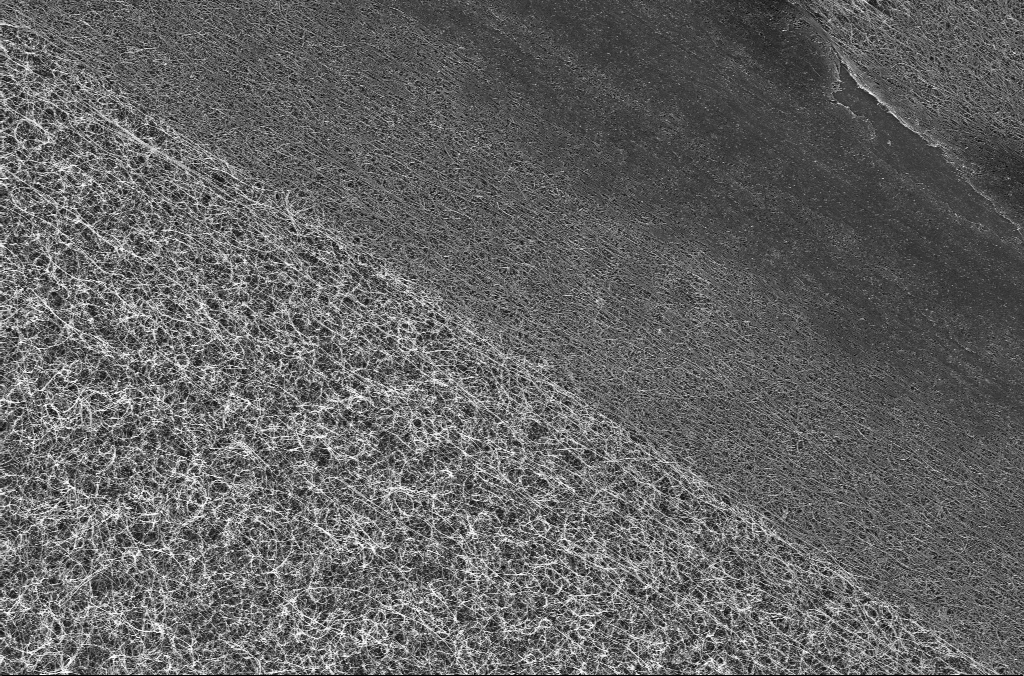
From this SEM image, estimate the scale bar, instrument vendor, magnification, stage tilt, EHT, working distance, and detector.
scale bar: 2000 nm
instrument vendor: Zeiss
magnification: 10 K X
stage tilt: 0°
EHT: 5 kV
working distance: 4.5 mm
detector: InLens